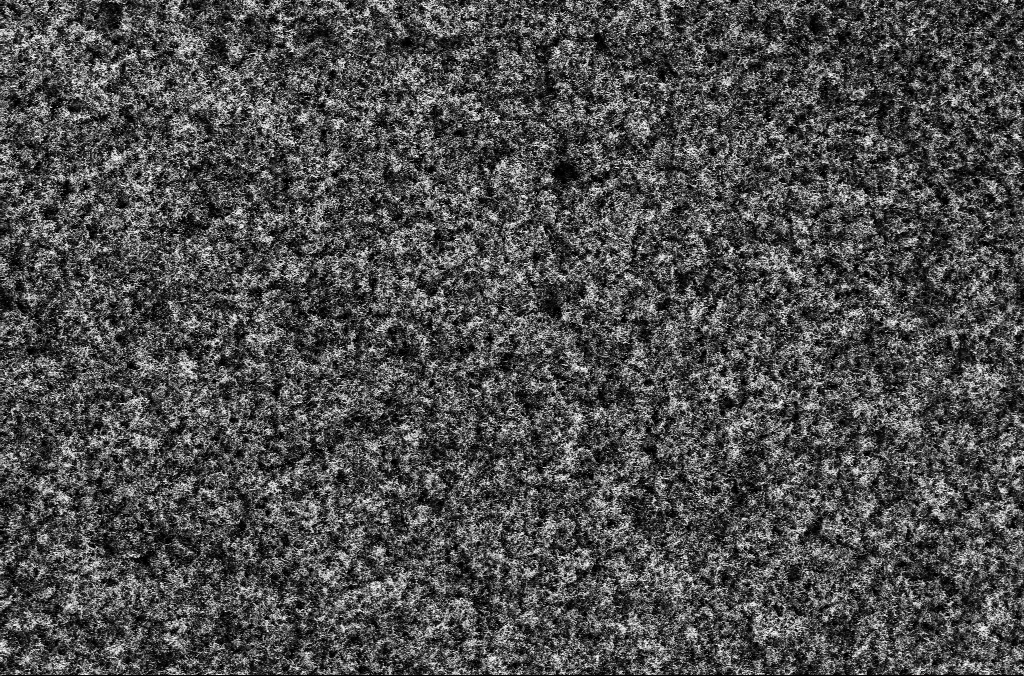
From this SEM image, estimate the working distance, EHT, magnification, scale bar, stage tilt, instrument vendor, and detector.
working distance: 5.3 mm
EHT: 1.8 kV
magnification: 2.5 K X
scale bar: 10000 nm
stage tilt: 0°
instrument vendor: Zeiss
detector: SE2